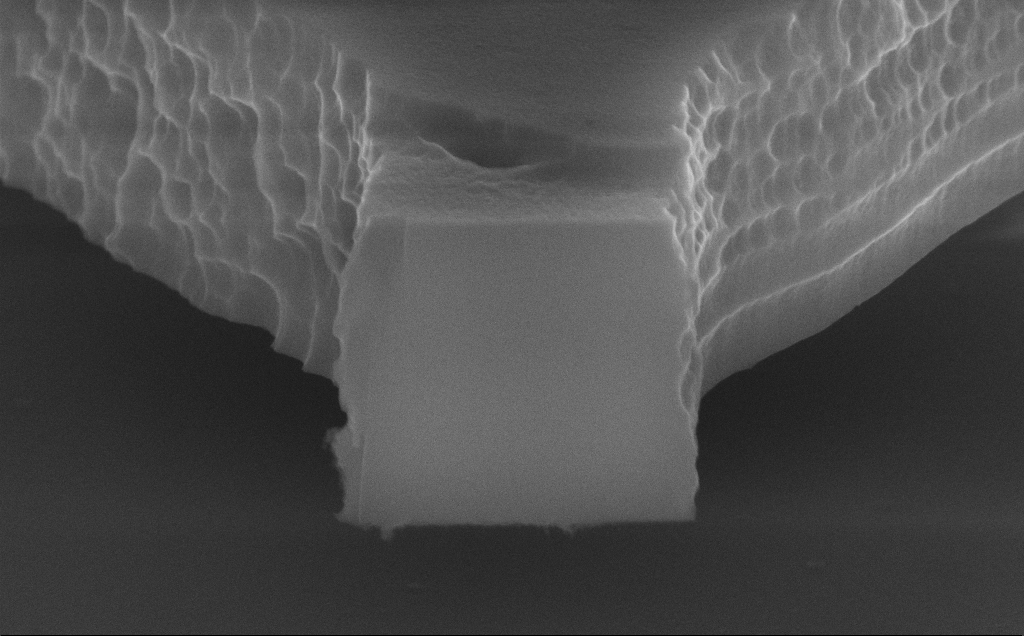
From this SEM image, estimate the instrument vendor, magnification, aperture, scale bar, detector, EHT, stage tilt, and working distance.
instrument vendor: Zeiss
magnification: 53.67 K X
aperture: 30 µm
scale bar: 1000 nm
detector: InLens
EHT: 10 kV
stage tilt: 70°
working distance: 9 mm